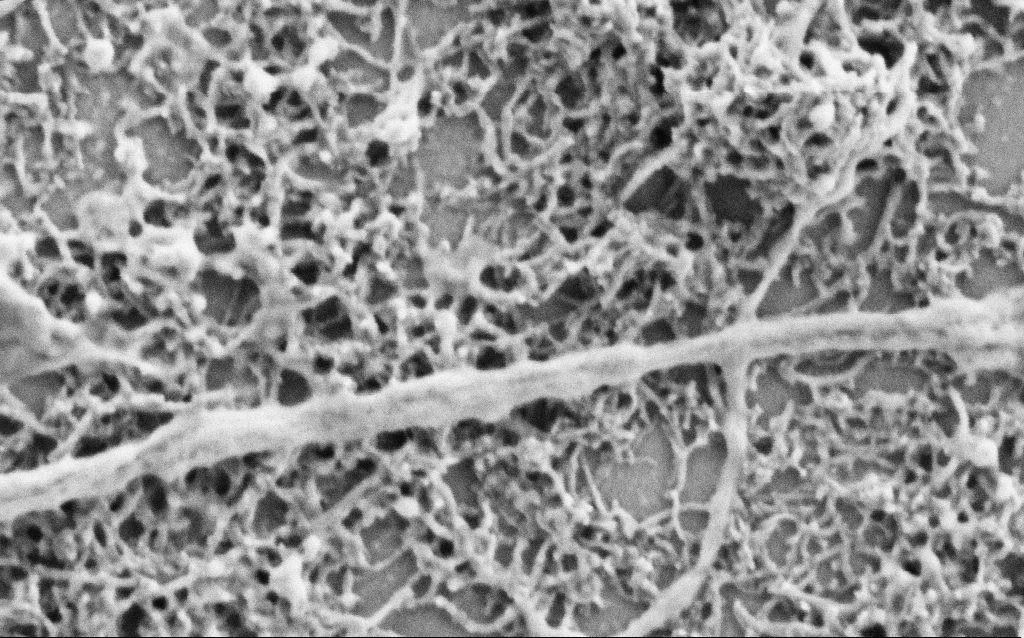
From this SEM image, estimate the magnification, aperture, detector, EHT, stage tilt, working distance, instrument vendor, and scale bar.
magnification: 75 K X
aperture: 30 µm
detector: SE2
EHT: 1.5 kV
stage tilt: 0°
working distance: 6.8 mm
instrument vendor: Zeiss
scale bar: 200 nm